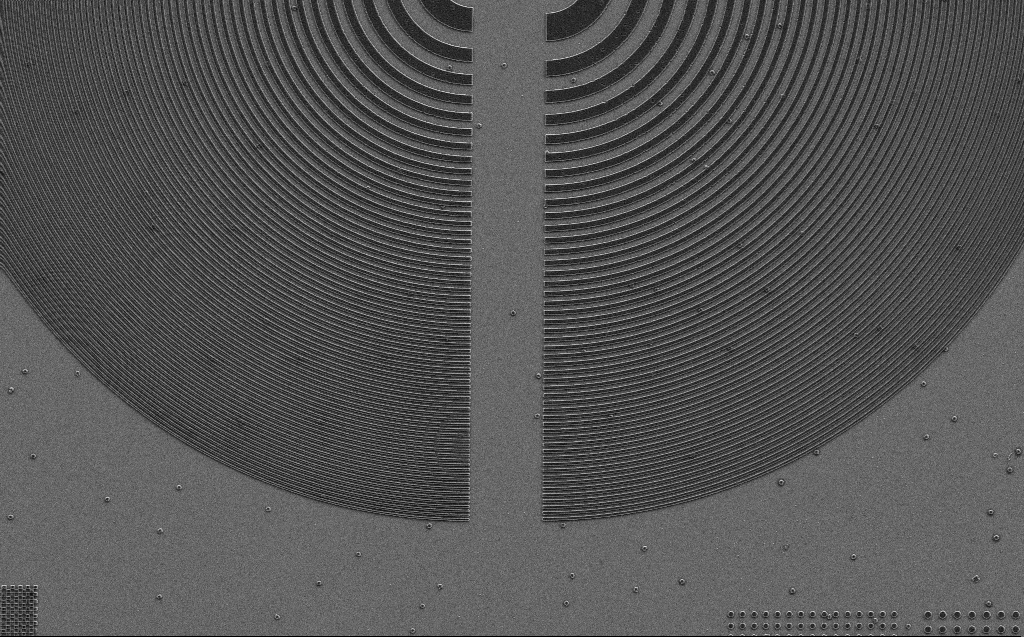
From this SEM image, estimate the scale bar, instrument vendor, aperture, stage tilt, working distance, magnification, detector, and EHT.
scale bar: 10000 nm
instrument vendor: Zeiss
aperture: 30 µm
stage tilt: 0°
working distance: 6 mm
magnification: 2.7 K X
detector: SE2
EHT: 3 kV